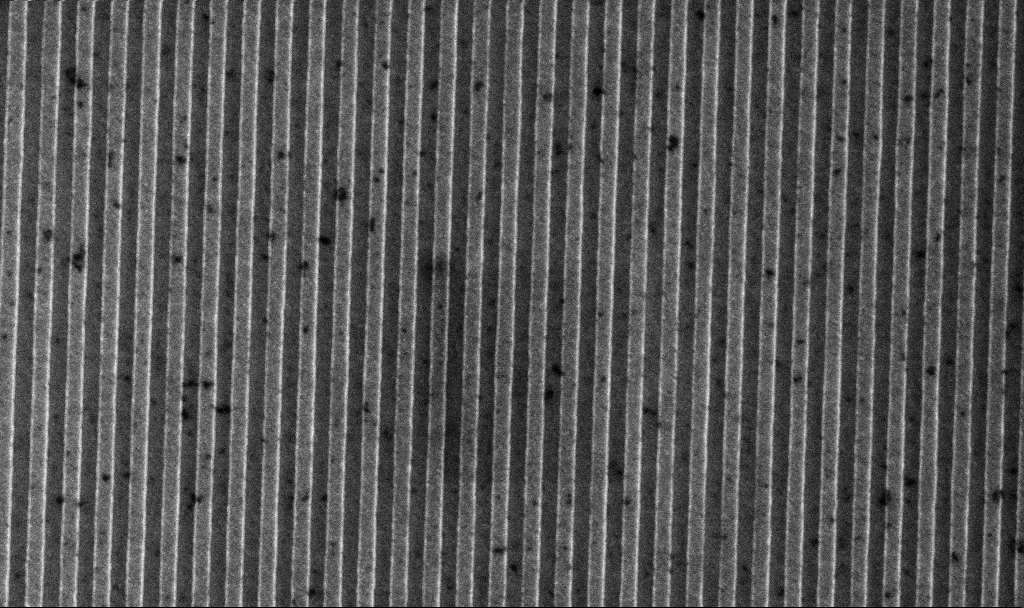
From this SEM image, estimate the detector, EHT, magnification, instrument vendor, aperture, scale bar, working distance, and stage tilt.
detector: InLens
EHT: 10 kV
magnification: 29.63 K X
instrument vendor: Zeiss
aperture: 60 µm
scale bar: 2000 nm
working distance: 5.6 mm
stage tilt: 0°